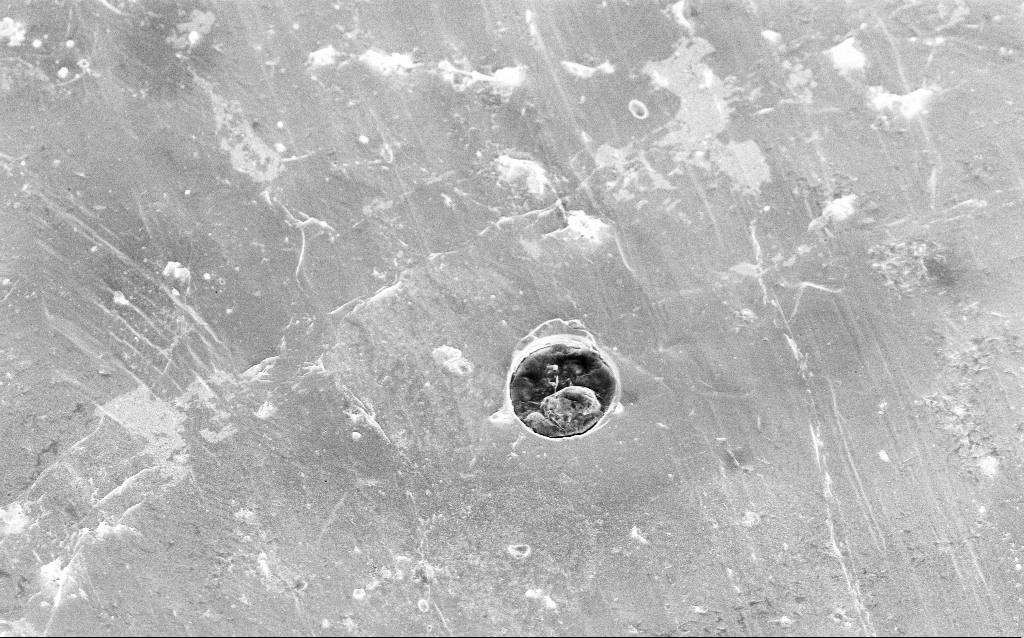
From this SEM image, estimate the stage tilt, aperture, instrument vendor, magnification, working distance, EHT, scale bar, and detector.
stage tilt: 25.7°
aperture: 30 µm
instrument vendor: Zeiss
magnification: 7.5 K X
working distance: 6.9 mm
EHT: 5 kV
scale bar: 2000 nm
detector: InLens